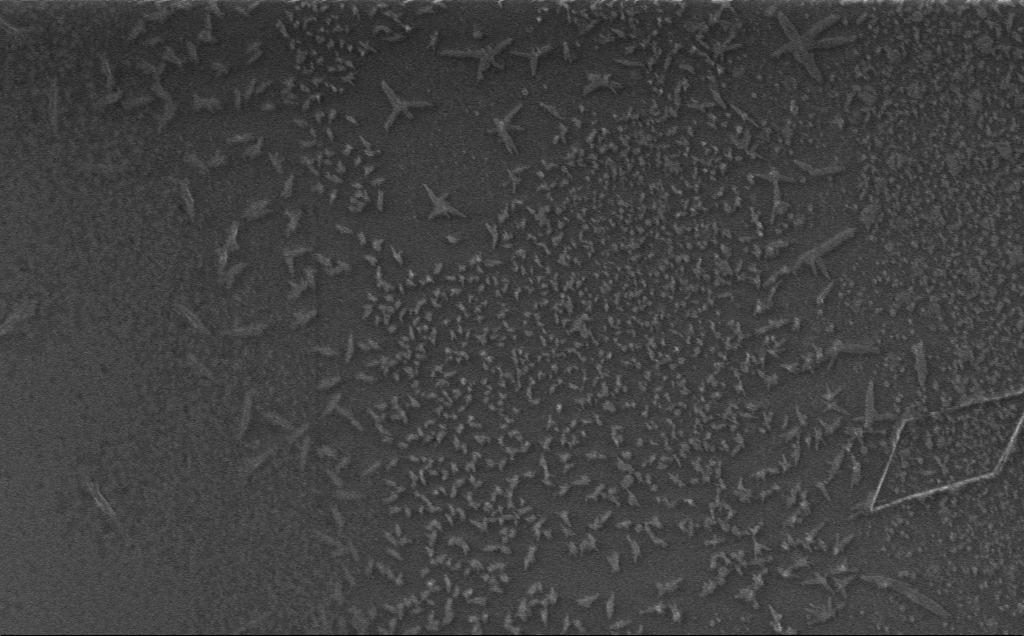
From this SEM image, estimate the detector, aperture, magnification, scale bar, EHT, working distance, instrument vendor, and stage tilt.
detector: InLens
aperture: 30 µm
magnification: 5.7 K X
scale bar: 10000 nm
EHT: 1 kV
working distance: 3 mm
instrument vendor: Zeiss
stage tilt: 0°